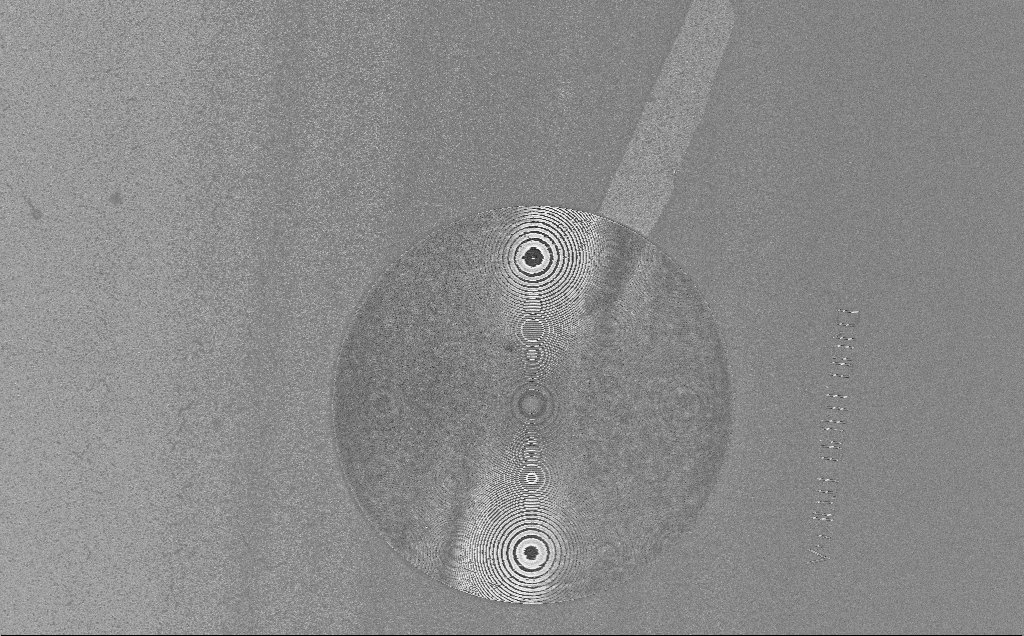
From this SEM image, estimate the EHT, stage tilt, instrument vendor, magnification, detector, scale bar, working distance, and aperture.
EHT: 5 kV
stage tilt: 0°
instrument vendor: Zeiss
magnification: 0.918 K X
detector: InLens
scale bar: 20000 nm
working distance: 10 mm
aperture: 30 µm